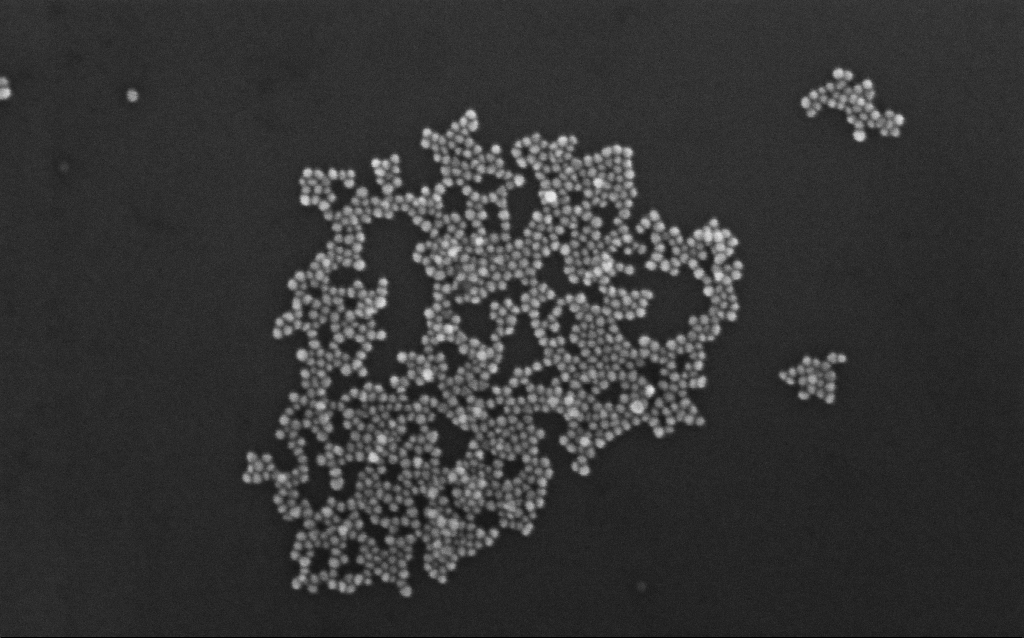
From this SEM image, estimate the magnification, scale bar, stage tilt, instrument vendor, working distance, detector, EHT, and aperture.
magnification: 223.46 K X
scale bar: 200 nm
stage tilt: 0°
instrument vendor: Zeiss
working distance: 7 mm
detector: InLens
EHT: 10 kV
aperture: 30 µm